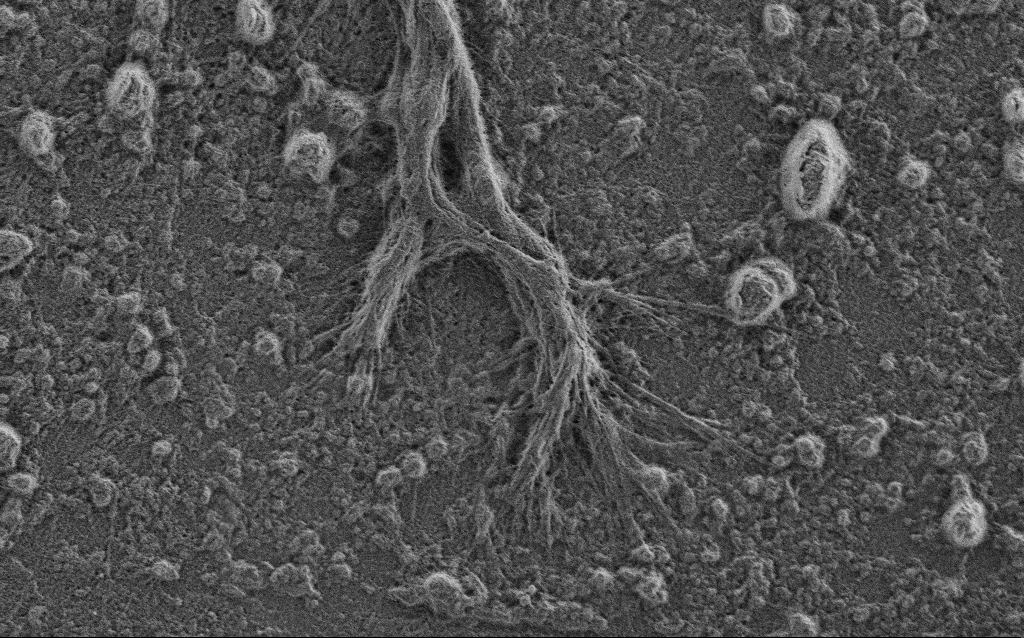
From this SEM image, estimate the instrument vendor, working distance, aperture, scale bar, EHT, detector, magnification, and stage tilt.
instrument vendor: Zeiss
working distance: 4 mm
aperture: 30 µm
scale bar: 2000 nm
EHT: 1 kV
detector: SE2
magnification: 7.5 K X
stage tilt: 0°